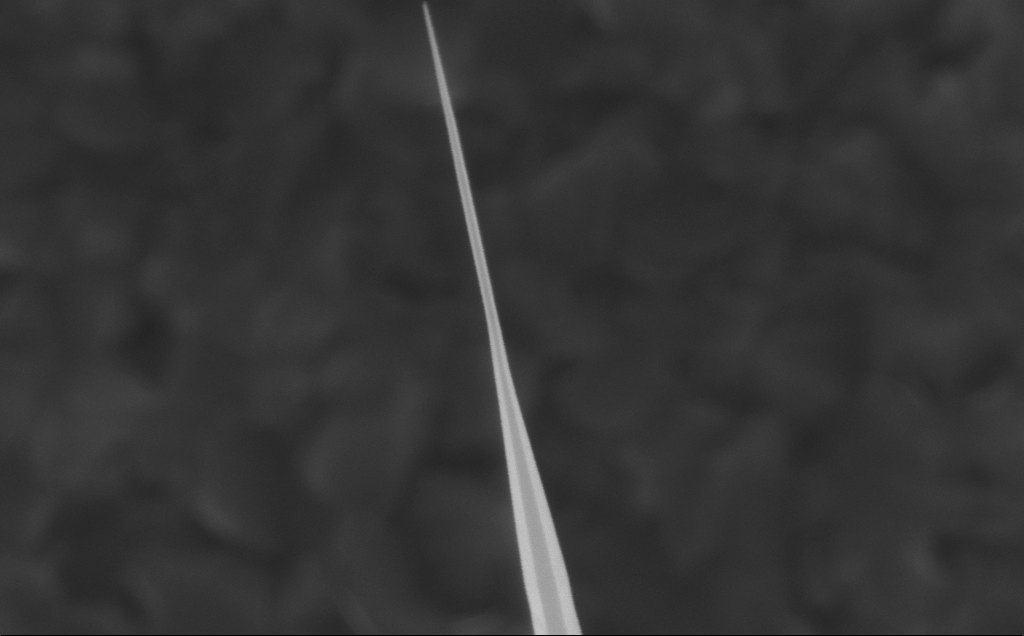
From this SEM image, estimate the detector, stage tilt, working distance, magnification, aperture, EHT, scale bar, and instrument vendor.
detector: InLens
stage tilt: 0°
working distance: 5 mm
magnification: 123.38 K X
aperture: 30 µm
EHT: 10 kV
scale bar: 200 nm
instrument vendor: Zeiss